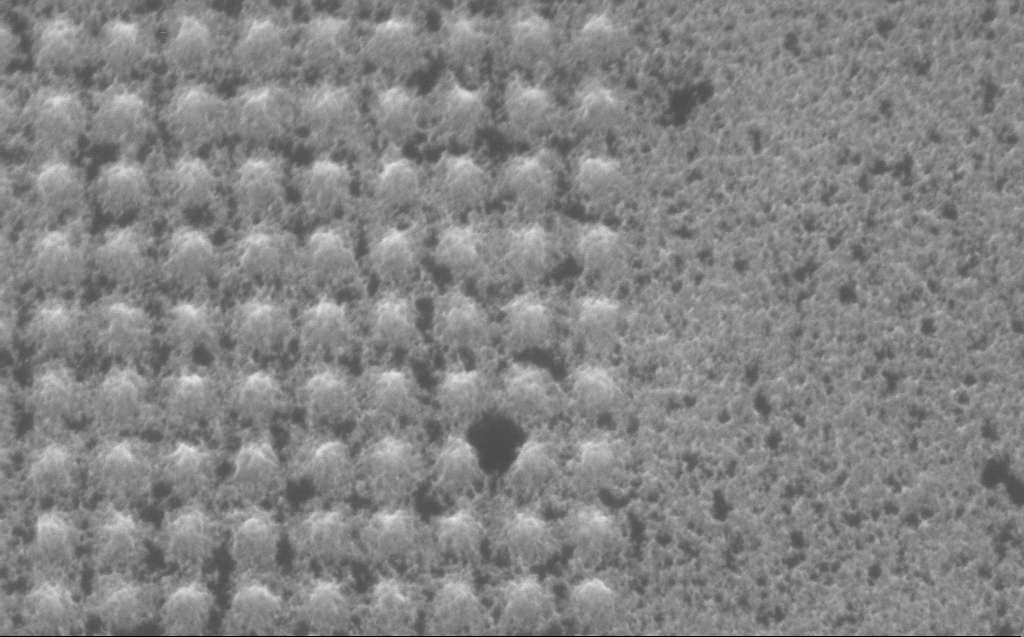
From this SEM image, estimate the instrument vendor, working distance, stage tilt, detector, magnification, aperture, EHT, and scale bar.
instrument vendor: Zeiss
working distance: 4 mm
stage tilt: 30°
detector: InLens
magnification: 125.55 K X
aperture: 30 µm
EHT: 5 kV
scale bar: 200 nm